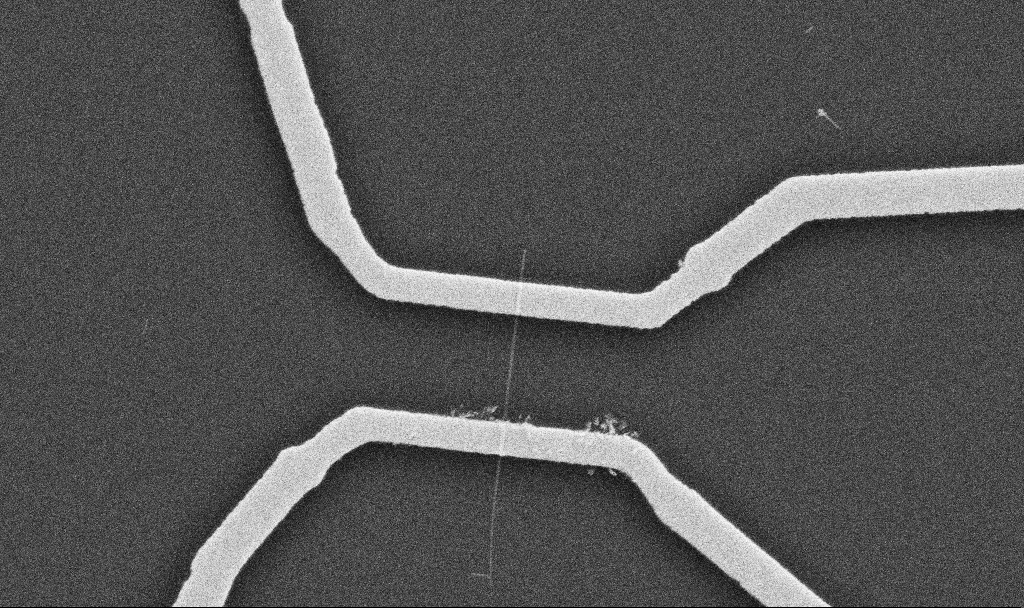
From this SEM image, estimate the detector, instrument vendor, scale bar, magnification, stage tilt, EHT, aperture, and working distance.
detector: SE2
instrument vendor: Zeiss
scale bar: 1000 nm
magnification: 20 K X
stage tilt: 0°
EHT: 10 kV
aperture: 30 µm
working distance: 10.7 mm